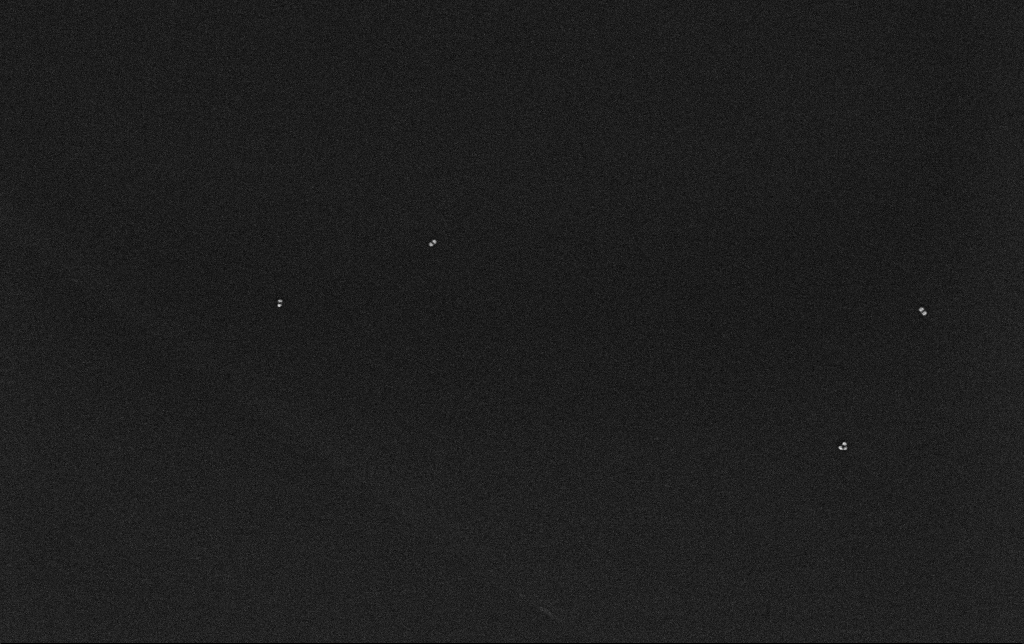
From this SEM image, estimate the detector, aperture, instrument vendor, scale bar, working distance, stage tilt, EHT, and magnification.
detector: InLens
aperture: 30 µm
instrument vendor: Zeiss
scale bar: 200 nm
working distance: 3.2 mm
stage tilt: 0°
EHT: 10 kV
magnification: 100 K X